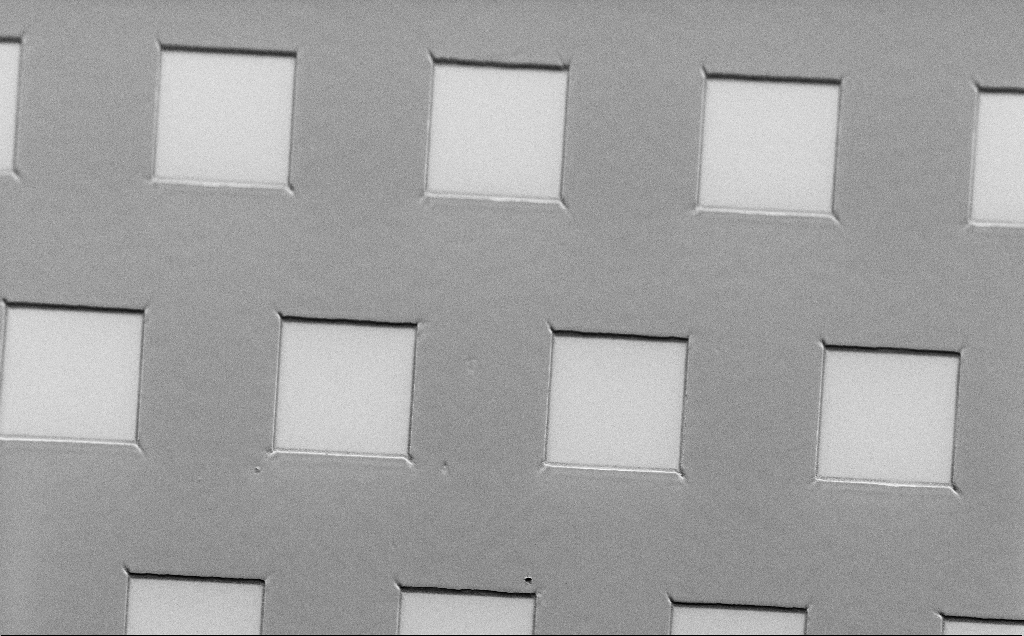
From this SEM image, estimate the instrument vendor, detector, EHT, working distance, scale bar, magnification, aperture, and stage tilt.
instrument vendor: Zeiss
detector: SE2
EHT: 1.5 kV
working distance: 7 mm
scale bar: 20000 nm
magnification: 0.997 K X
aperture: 30 µm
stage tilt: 45°